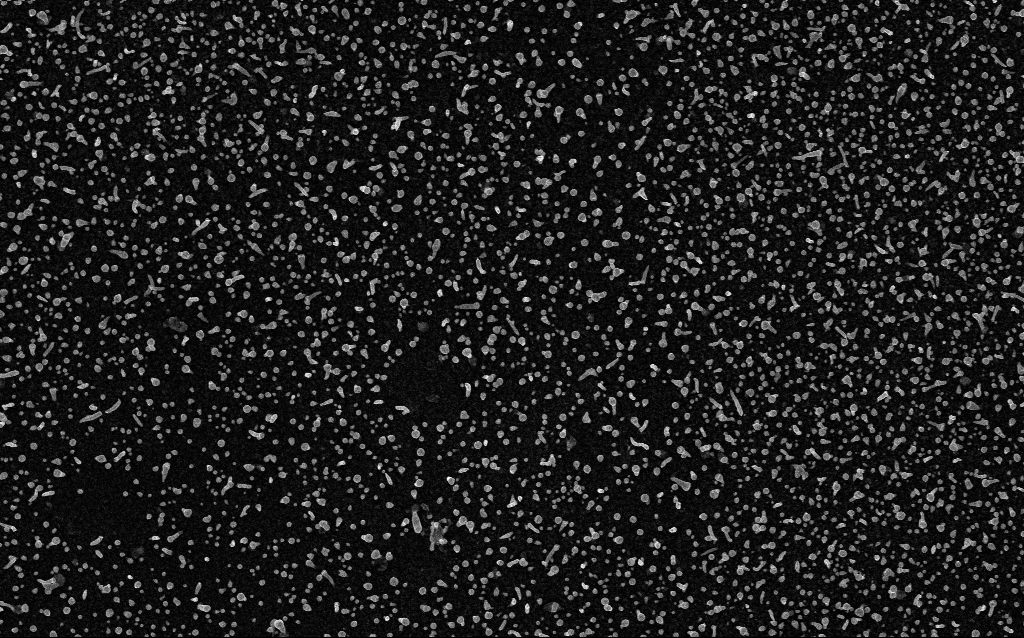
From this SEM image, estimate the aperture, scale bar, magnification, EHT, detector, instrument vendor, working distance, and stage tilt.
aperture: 30 µm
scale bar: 1000 nm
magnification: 20 K X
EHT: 5 kV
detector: InLens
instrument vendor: Zeiss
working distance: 2.1 mm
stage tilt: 0°